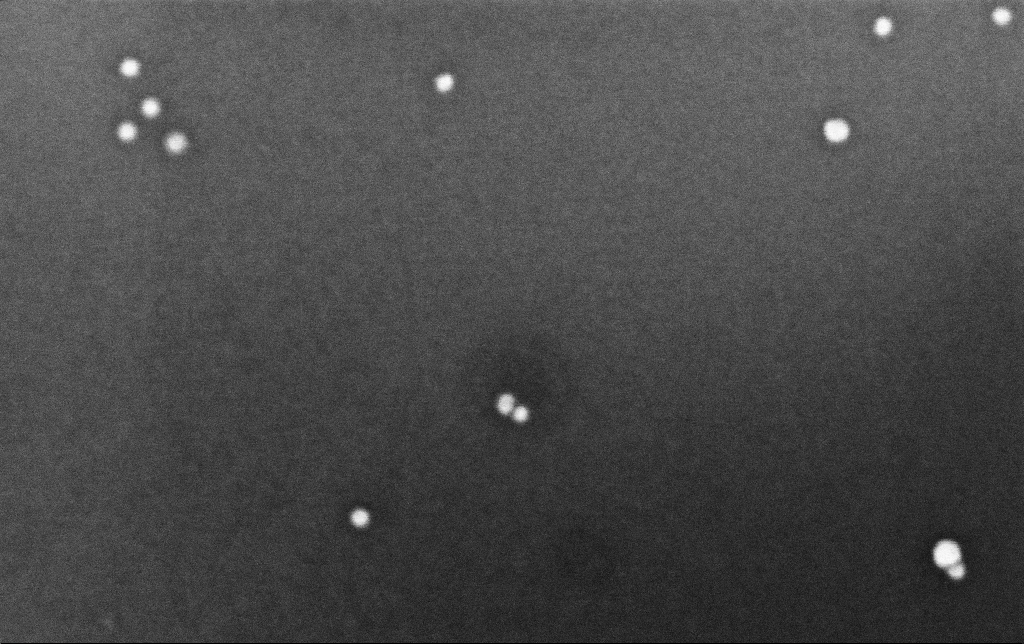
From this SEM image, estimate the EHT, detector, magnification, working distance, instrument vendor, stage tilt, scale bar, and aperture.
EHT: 8 kV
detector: InLens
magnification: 400 K X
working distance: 6.6 mm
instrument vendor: Zeiss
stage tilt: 0°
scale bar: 100 nm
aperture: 30 µm